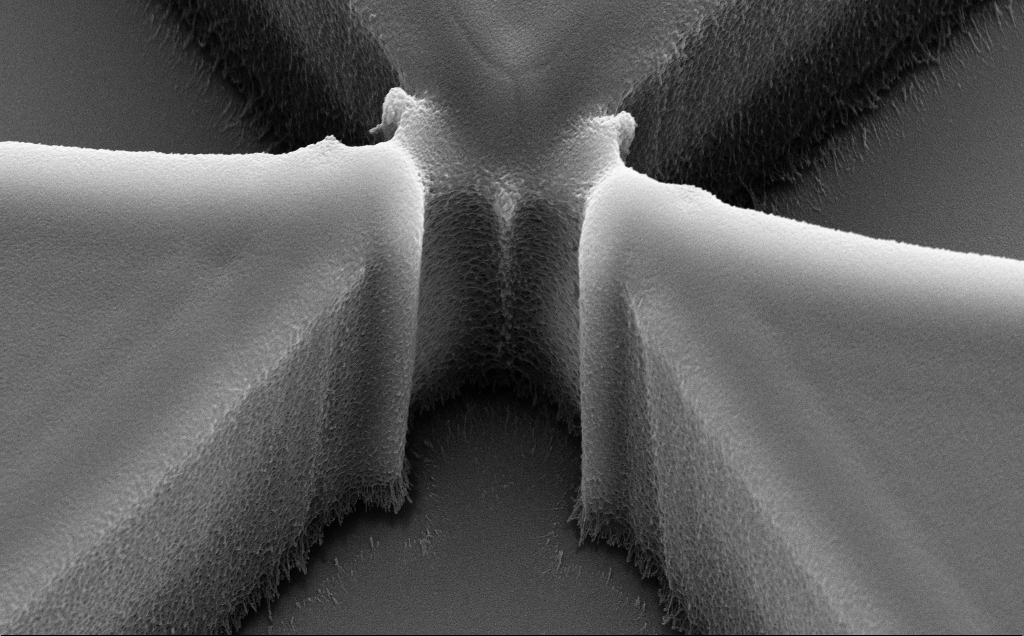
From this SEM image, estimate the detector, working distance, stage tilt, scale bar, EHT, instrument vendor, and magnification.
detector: SE2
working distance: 11 mm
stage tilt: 35.3°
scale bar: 2000 nm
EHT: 10 kV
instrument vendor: Zeiss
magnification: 11.15 K X